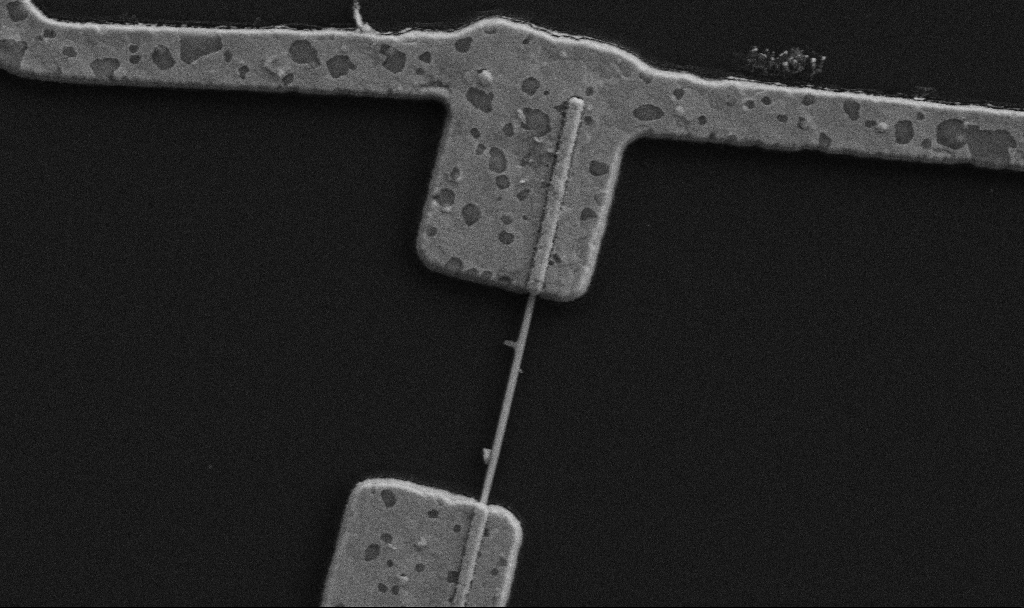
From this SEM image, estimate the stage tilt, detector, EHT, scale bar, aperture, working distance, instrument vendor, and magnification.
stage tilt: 0°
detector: SE2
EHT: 5 kV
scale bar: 2000 nm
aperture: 30 µm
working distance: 8.7 mm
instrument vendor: Zeiss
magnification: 30 K X